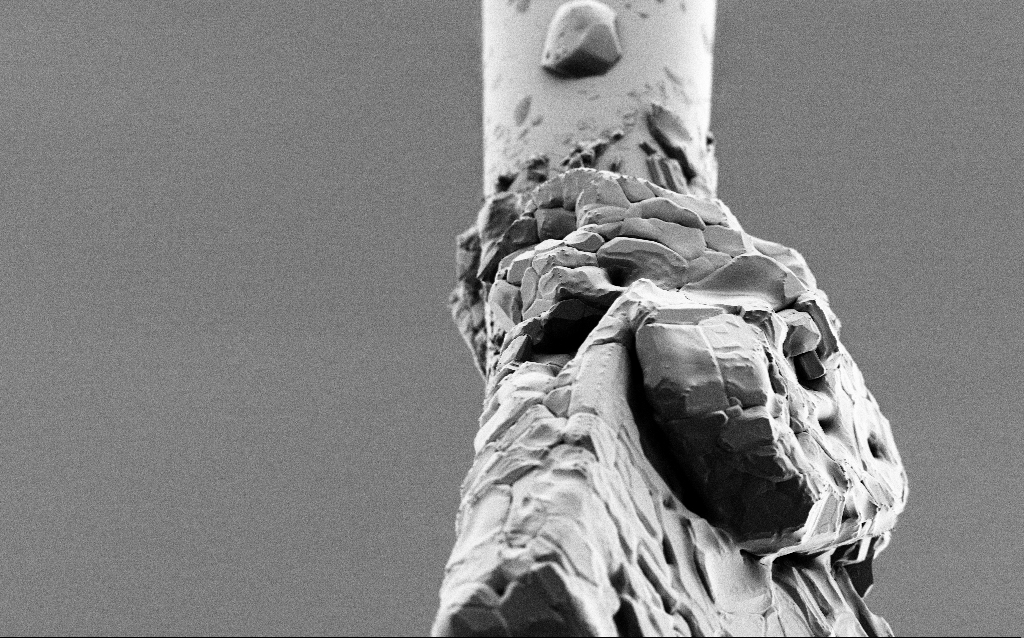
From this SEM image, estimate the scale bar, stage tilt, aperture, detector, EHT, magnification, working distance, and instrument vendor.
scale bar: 10000 nm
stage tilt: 45°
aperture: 30 µm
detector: SE2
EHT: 1 kV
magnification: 2.5 K X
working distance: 6.4 mm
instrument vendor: Zeiss